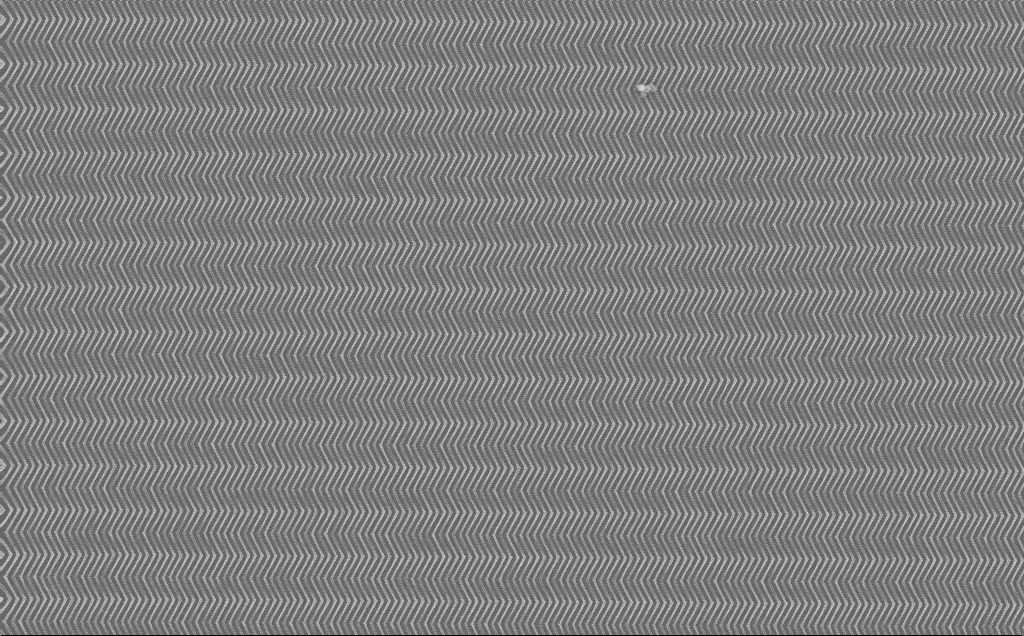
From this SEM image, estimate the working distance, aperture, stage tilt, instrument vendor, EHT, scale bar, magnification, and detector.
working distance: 7 mm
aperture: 30 µm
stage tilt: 0°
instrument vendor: Zeiss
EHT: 10 kV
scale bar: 2000 nm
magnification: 8.26 K X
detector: InLens